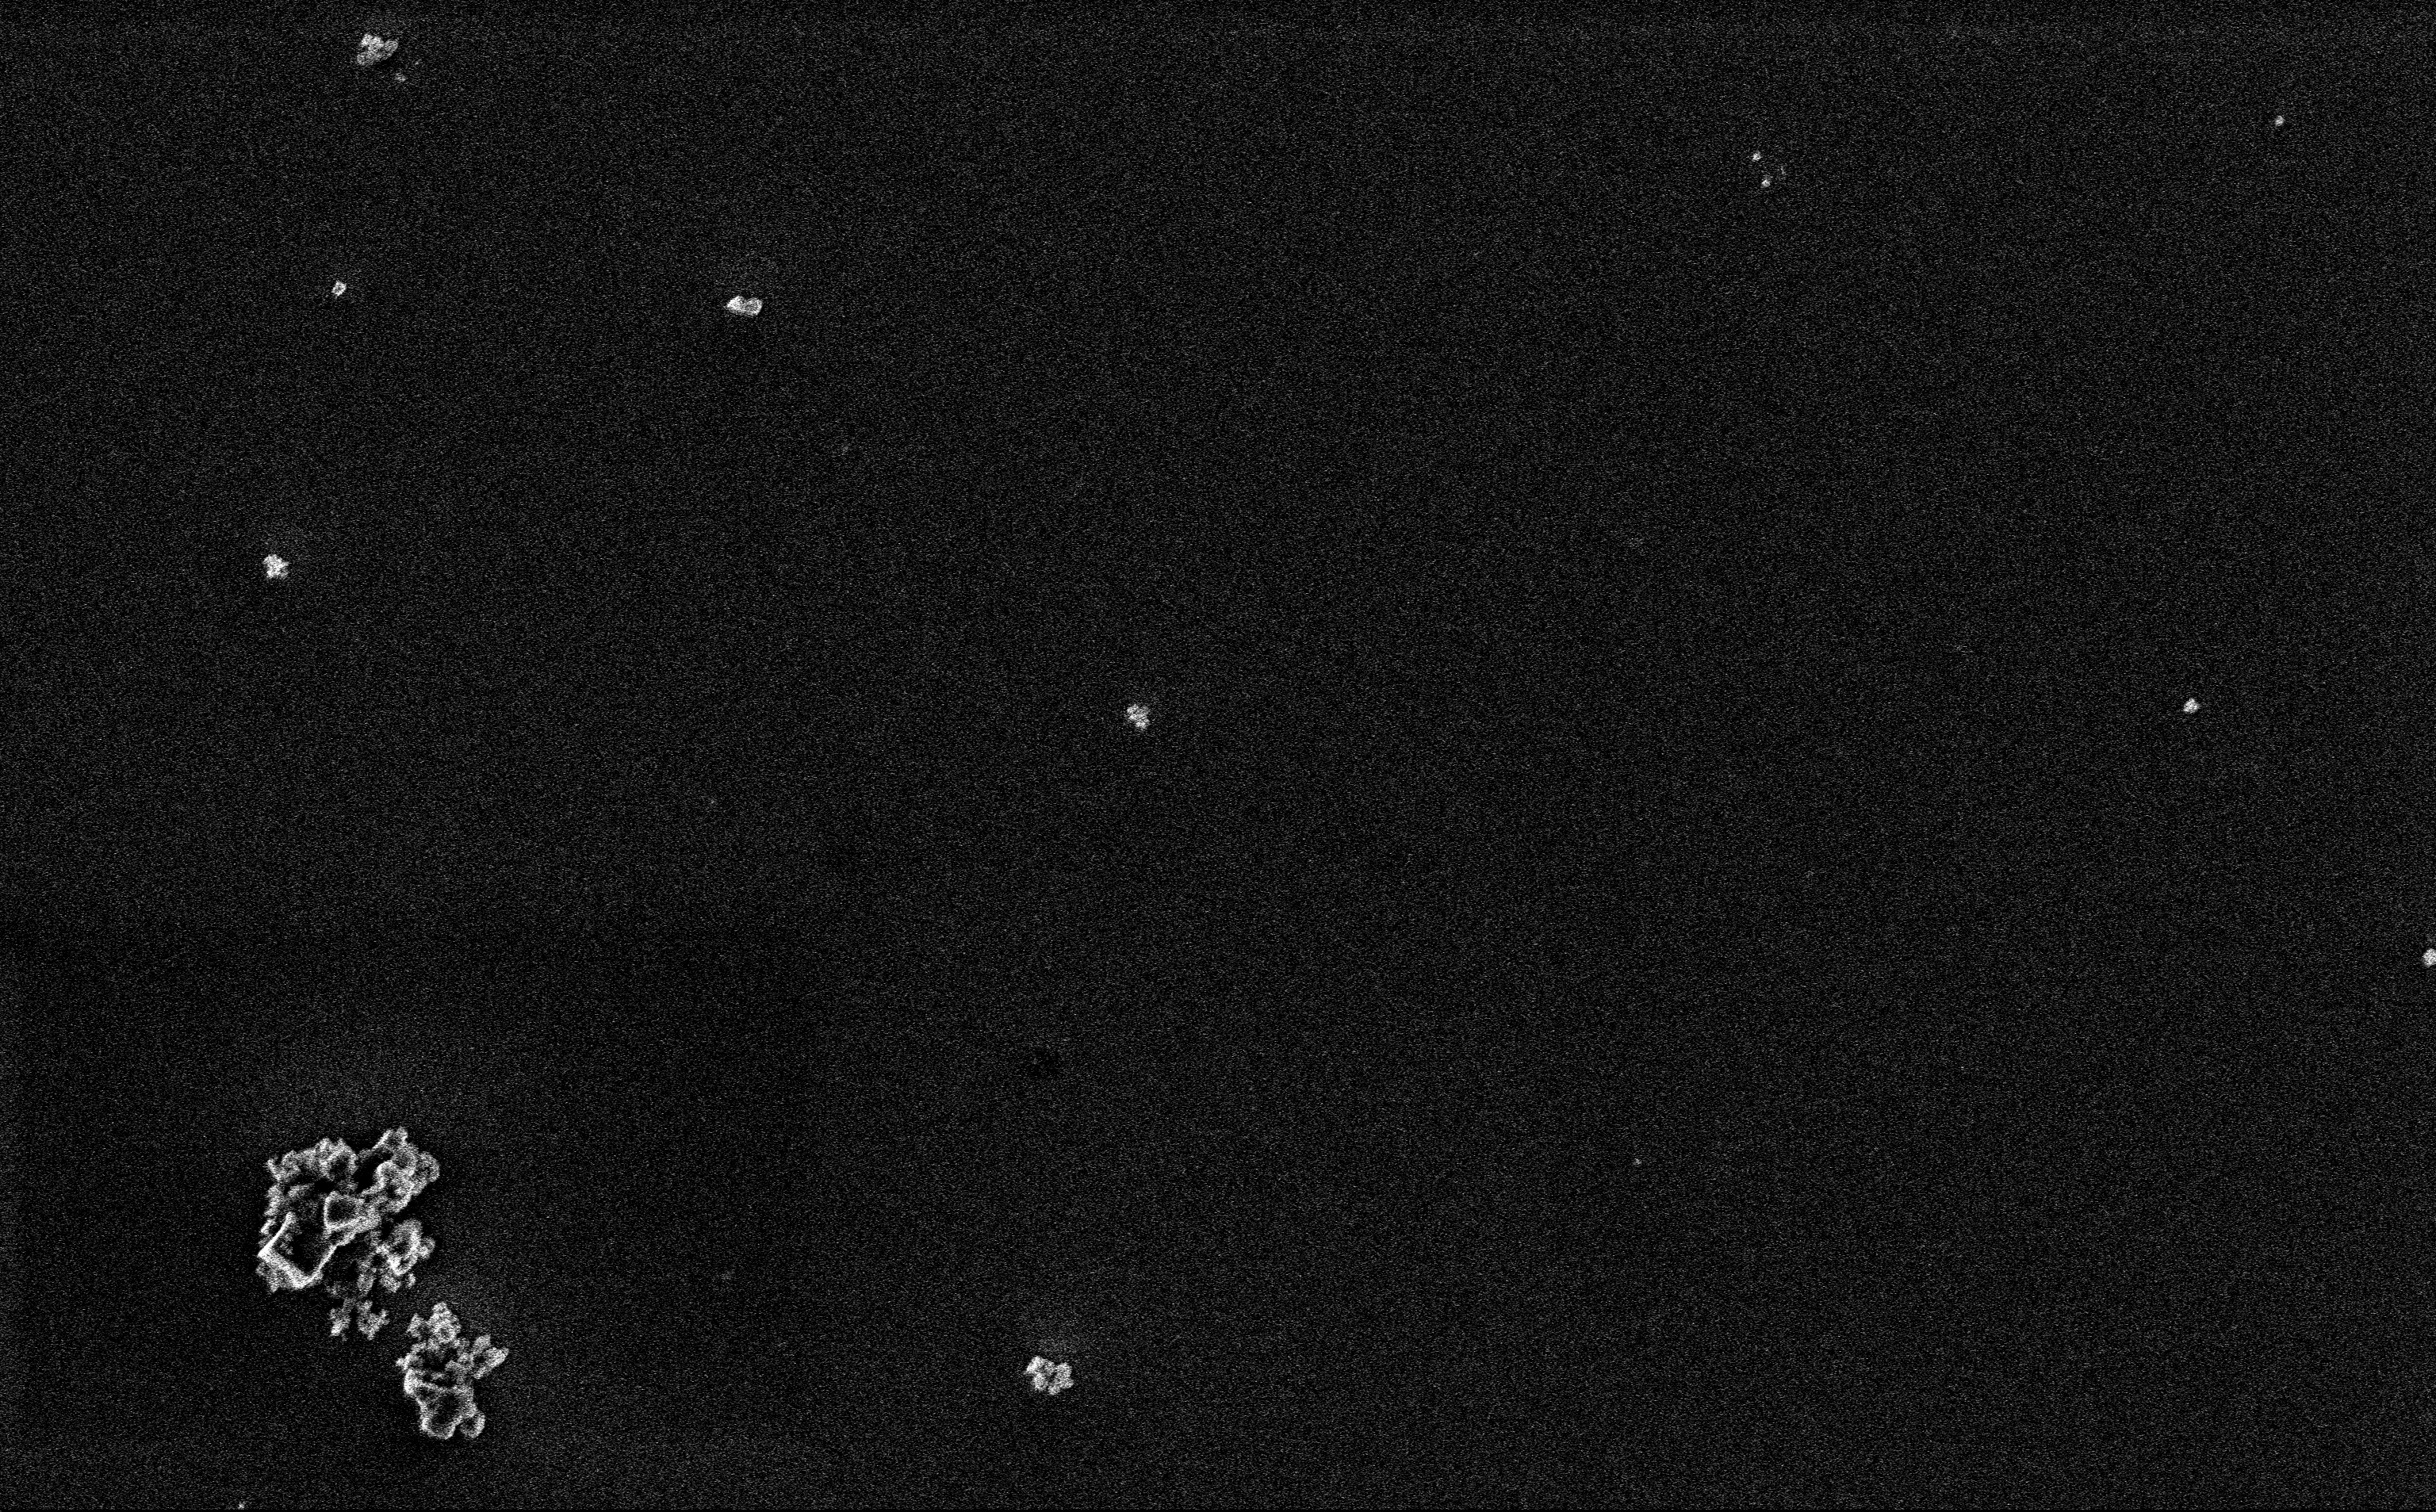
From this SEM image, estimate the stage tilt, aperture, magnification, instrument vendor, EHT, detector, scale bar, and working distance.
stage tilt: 0°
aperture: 30 µm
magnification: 12.85 K X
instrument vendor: Zeiss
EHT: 3 kV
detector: InLens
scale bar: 2000 nm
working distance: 3 mm